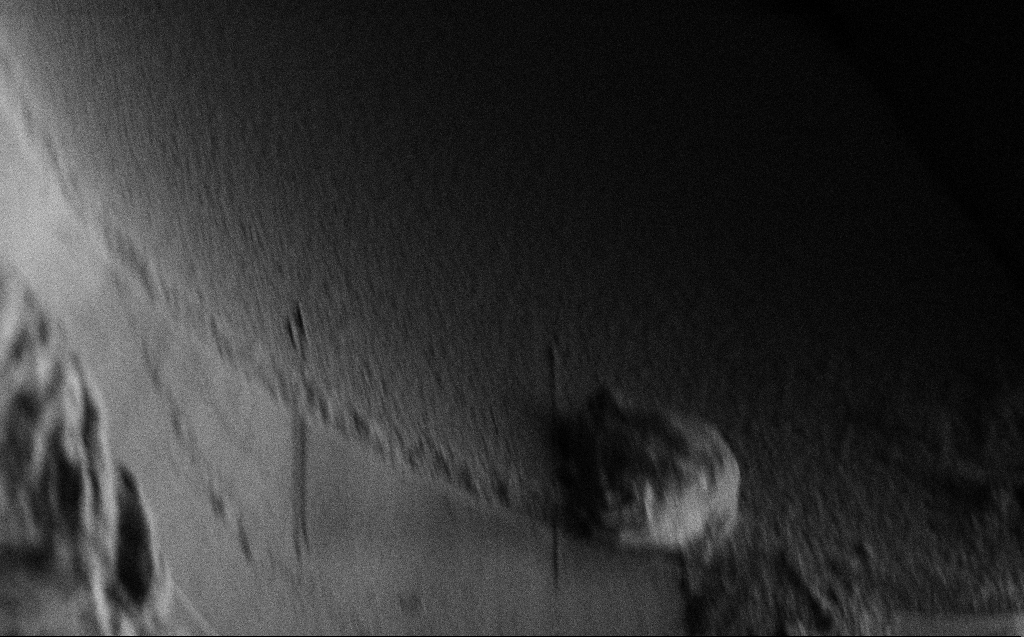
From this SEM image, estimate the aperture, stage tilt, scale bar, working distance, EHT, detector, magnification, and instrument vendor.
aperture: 30 µm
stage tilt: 45°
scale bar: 2000 nm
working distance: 3 mm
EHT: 1 kV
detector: SE2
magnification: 23.53 K X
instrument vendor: Zeiss